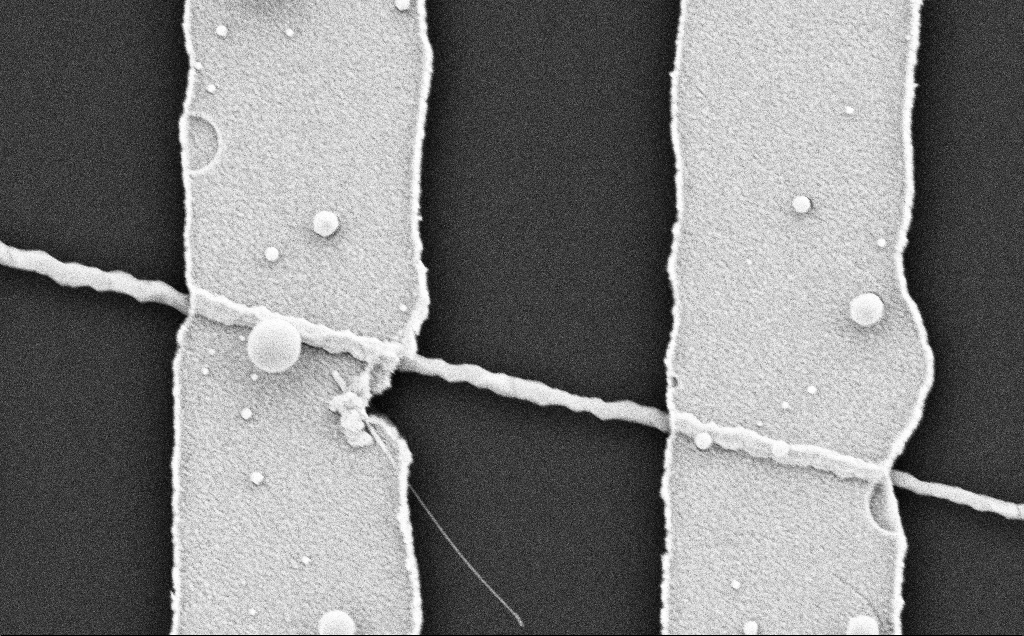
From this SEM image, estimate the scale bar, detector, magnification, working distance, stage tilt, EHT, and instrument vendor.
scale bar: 1000 nm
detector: SE2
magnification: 45.15 K X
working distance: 8 mm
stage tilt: -0.7°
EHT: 5 kV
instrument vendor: Zeiss